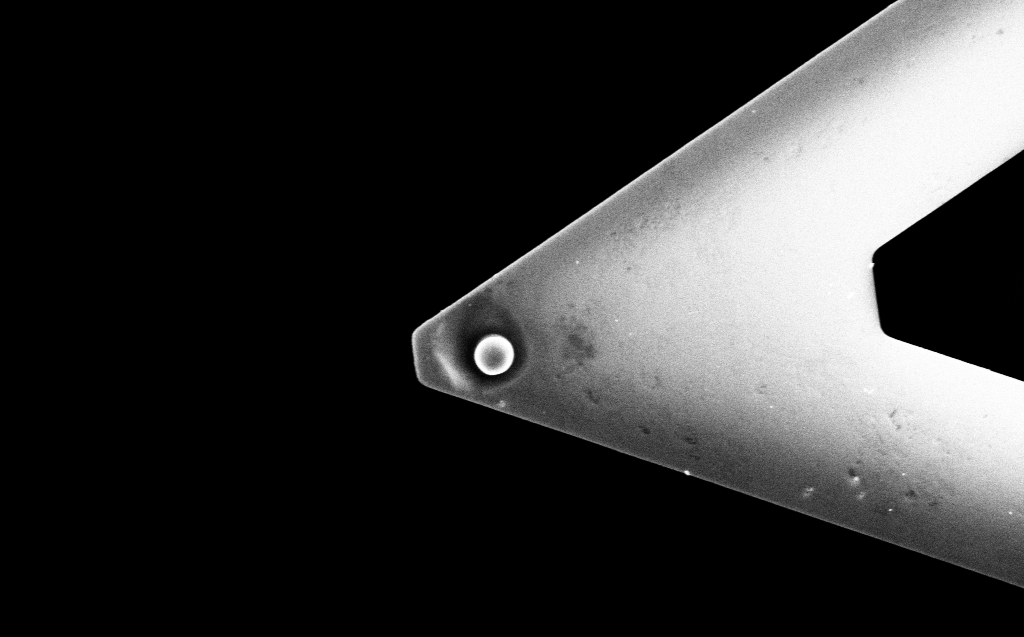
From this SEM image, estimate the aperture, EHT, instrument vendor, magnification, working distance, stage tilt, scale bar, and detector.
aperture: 30 µm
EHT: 10 kV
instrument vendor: Zeiss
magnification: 3.16 K X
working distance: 19 mm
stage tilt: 0°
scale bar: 20000 nm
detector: SE2